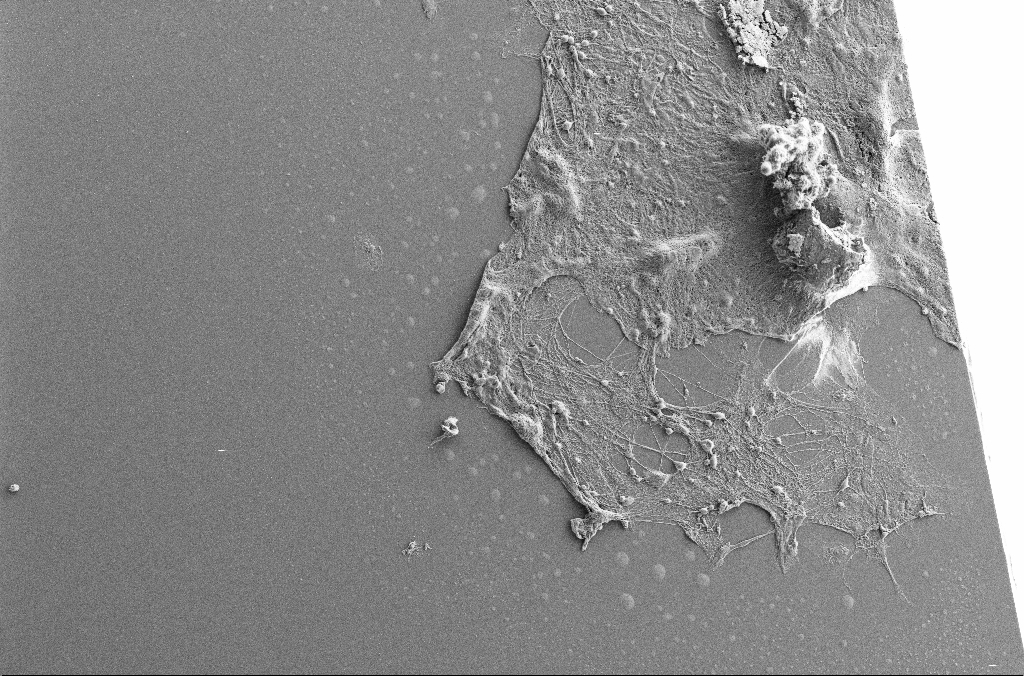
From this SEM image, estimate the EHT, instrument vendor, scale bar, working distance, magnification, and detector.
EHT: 7 kV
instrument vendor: Zeiss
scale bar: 100000 nm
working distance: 3.8 mm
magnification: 0.25 K X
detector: SE2